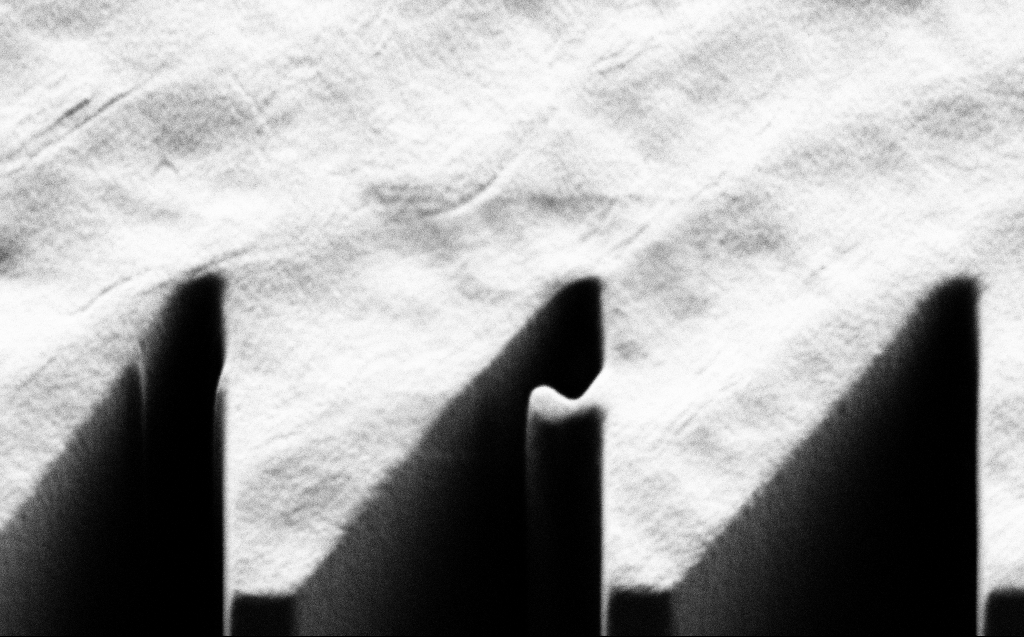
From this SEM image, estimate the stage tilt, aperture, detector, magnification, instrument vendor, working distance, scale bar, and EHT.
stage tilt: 45°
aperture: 30 µm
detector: SE2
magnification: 12.18 K X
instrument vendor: Zeiss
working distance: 6 mm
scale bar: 2000 nm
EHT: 1 kV